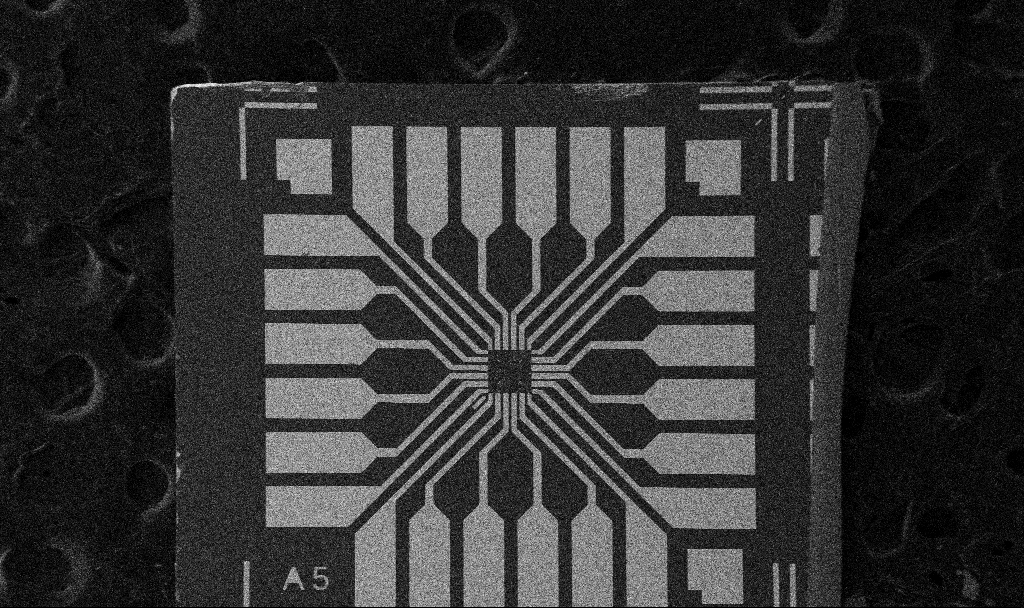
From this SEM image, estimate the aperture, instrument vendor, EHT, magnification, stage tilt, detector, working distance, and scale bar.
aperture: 30 µm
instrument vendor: Zeiss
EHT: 5 kV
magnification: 0.1 K X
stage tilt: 0°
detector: SE2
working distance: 10.7 mm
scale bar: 200000 nm